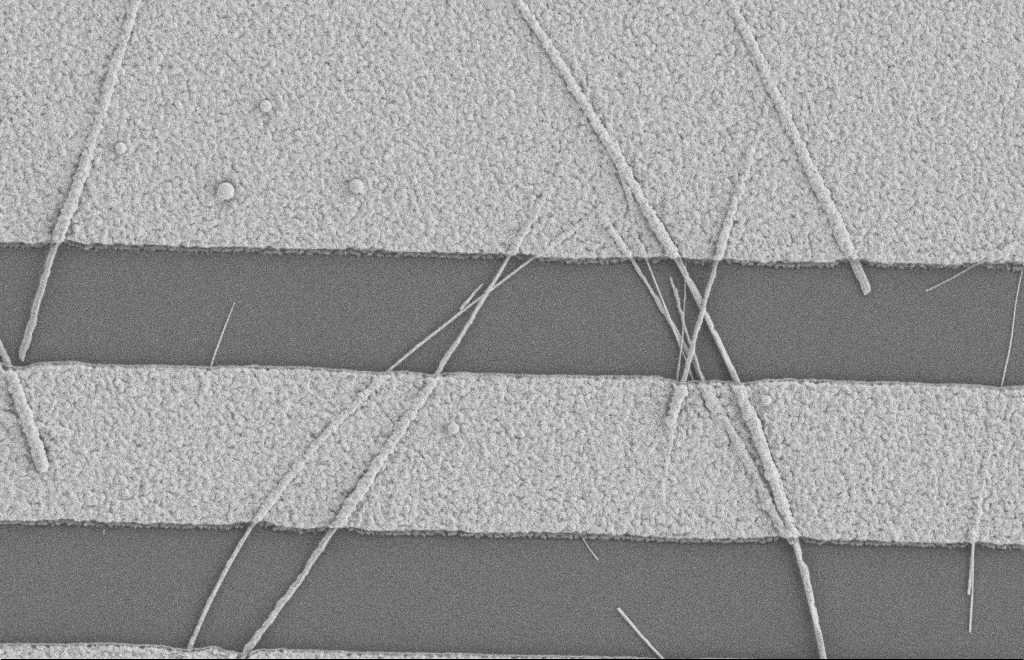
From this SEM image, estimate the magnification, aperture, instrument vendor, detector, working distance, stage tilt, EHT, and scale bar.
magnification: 16.9 K X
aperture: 20 µm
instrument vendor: Zeiss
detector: SE2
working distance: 8 mm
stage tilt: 0°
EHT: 2 kV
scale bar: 2000 nm